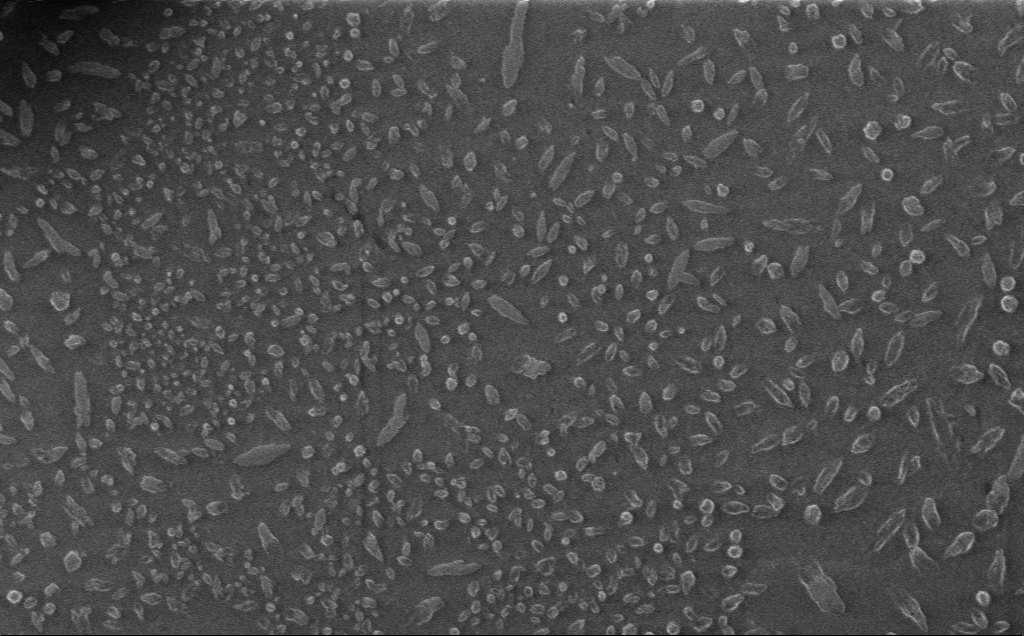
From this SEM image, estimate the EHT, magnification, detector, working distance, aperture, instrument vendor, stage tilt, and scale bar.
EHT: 1 kV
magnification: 7.27 K X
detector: InLens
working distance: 3 mm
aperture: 30 µm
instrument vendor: Zeiss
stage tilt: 0°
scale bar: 2000 nm